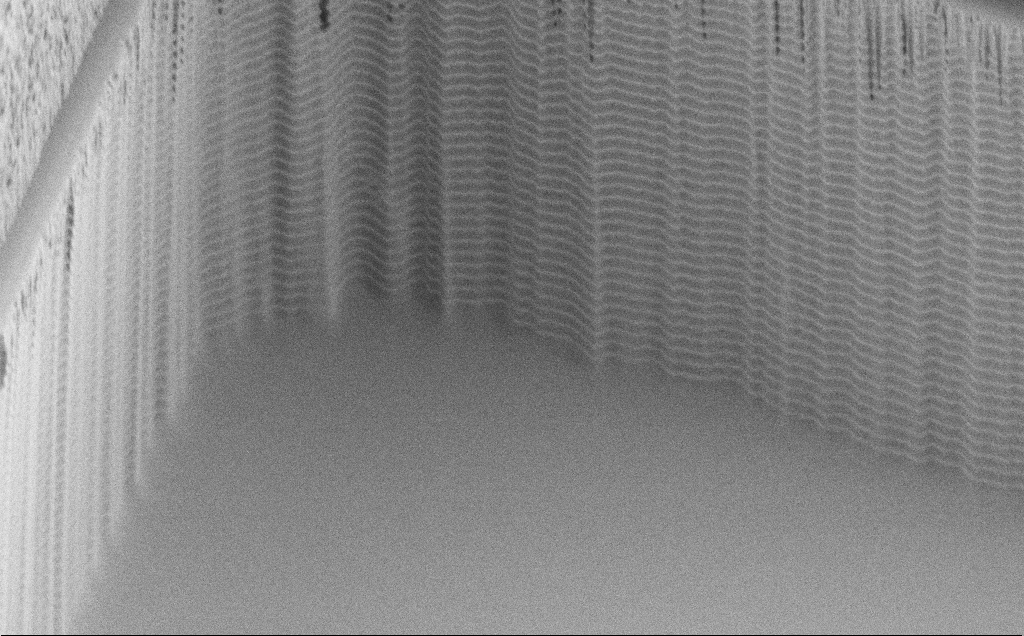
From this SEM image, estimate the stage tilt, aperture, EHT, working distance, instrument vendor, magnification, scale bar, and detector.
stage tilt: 45°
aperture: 30 µm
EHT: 1.2 kV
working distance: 8 mm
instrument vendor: Zeiss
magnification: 12.86 K X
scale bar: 2000 nm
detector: SE2